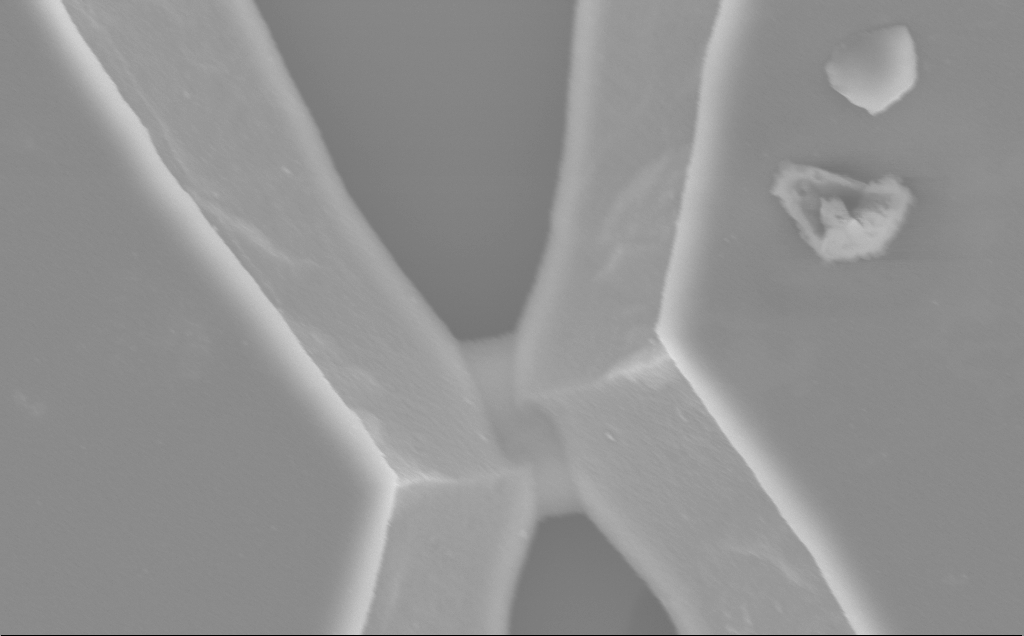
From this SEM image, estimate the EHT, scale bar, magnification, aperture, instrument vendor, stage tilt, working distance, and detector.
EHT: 5 kV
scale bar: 1000 nm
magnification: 37.92 K X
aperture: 30 µm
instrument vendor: Zeiss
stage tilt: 0°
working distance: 10 mm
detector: InLens